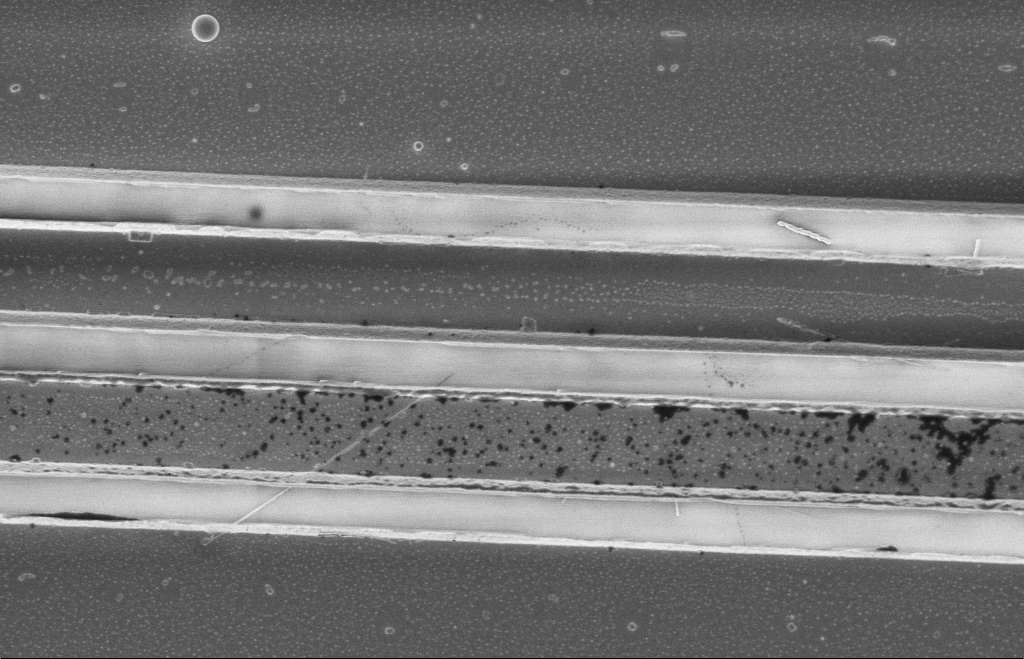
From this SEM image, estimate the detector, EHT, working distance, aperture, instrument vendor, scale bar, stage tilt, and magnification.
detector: InLens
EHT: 5 kV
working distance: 12 mm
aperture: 20 µm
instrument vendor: Zeiss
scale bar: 2000 nm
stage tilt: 0°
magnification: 9.01 K X